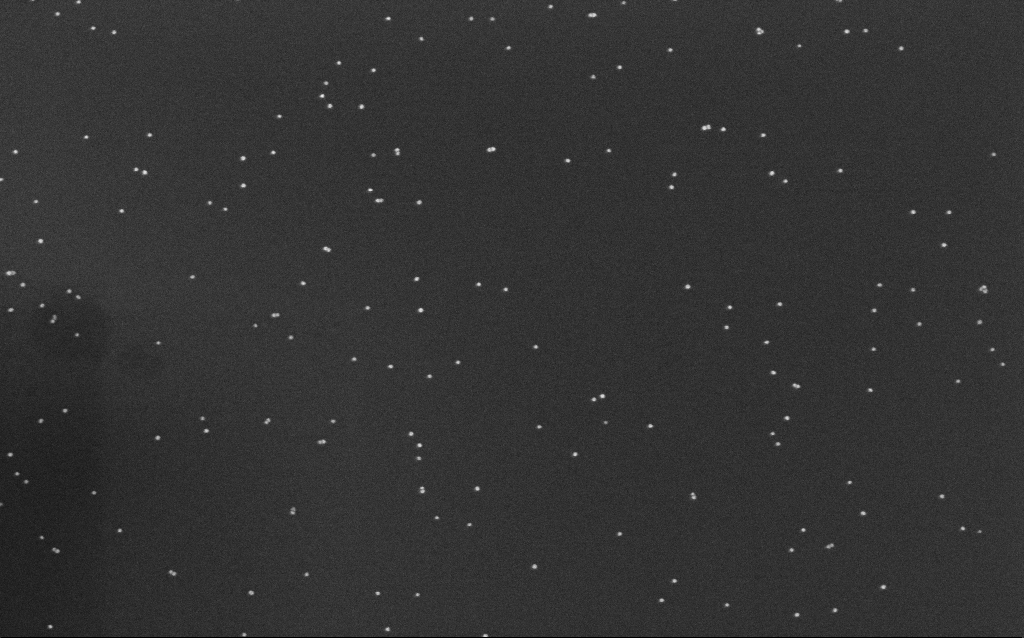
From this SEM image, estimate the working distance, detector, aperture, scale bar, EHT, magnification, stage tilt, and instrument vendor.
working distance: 6.6 mm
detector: InLens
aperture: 30 µm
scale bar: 200 nm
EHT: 10 kV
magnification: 100 K X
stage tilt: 0°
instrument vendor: Zeiss